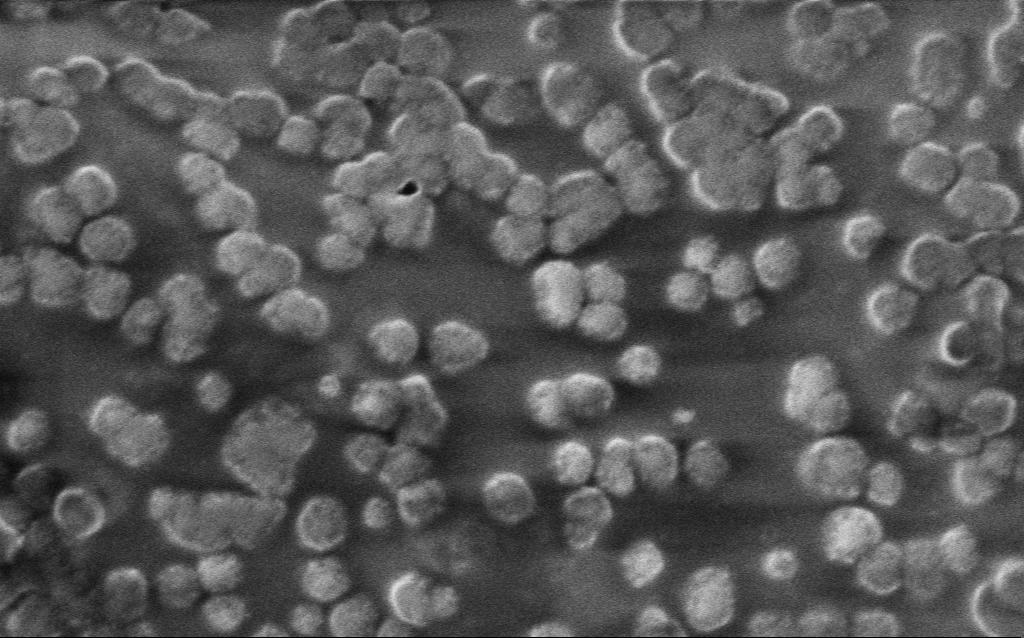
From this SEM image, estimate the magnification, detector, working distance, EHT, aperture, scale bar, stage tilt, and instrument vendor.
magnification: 106.96 K X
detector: InLens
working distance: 4 mm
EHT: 1 kV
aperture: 30 µm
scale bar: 200 nm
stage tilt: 0°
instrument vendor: Zeiss